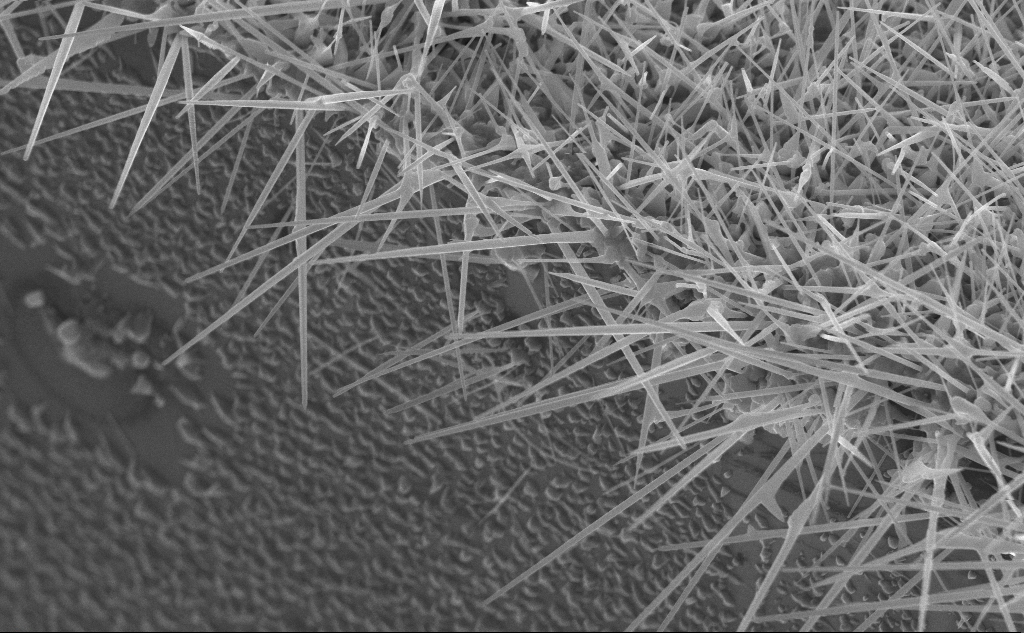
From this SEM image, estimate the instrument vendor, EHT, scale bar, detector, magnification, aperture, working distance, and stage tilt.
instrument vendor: Zeiss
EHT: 10 kV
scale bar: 2000 nm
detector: InLens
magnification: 25.31 K X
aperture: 30 µm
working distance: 4 mm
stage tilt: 45°